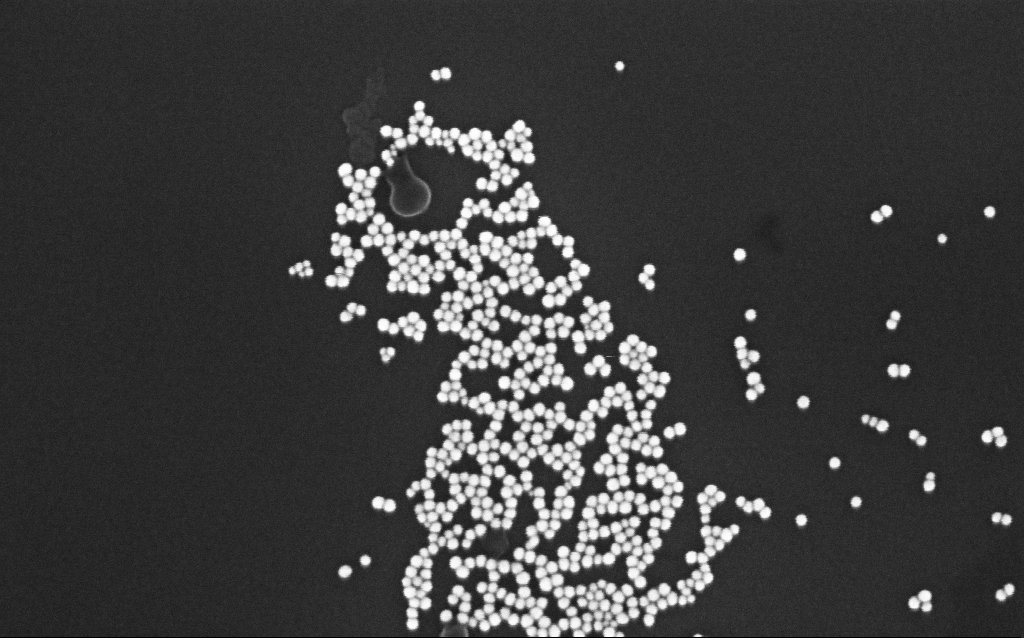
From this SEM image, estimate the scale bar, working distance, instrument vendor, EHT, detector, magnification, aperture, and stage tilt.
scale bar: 200 nm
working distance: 3.3 mm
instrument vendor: Zeiss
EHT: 5 kV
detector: InLens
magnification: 200 K X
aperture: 30 µm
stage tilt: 0°